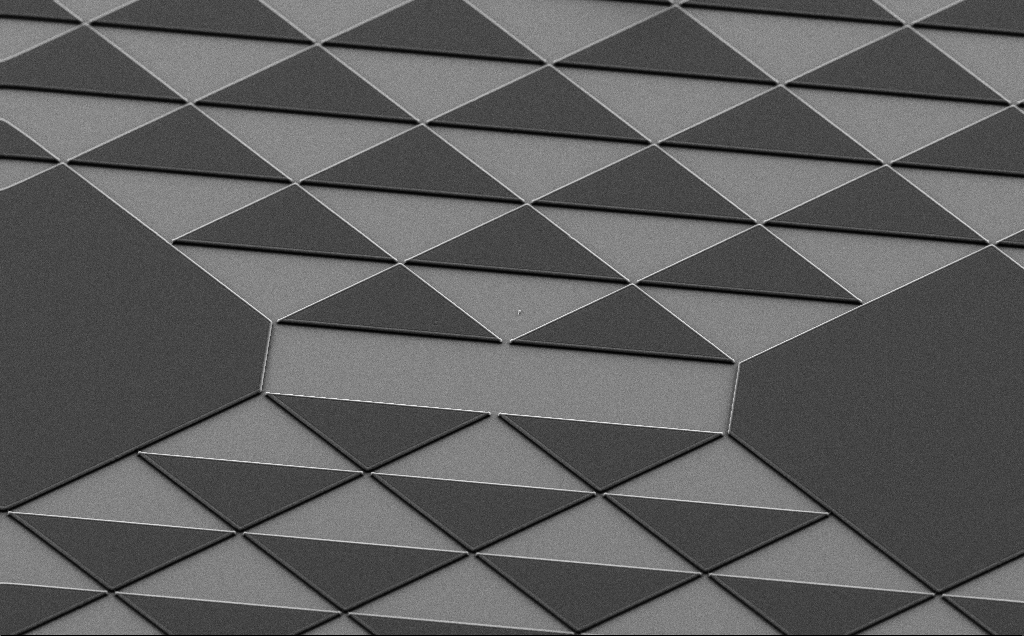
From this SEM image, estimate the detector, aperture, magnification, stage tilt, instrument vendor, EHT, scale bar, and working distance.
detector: SE2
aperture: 30 µm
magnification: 0.926 K X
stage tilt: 35°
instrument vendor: Zeiss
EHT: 7 kV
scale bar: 20000 nm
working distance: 5 mm